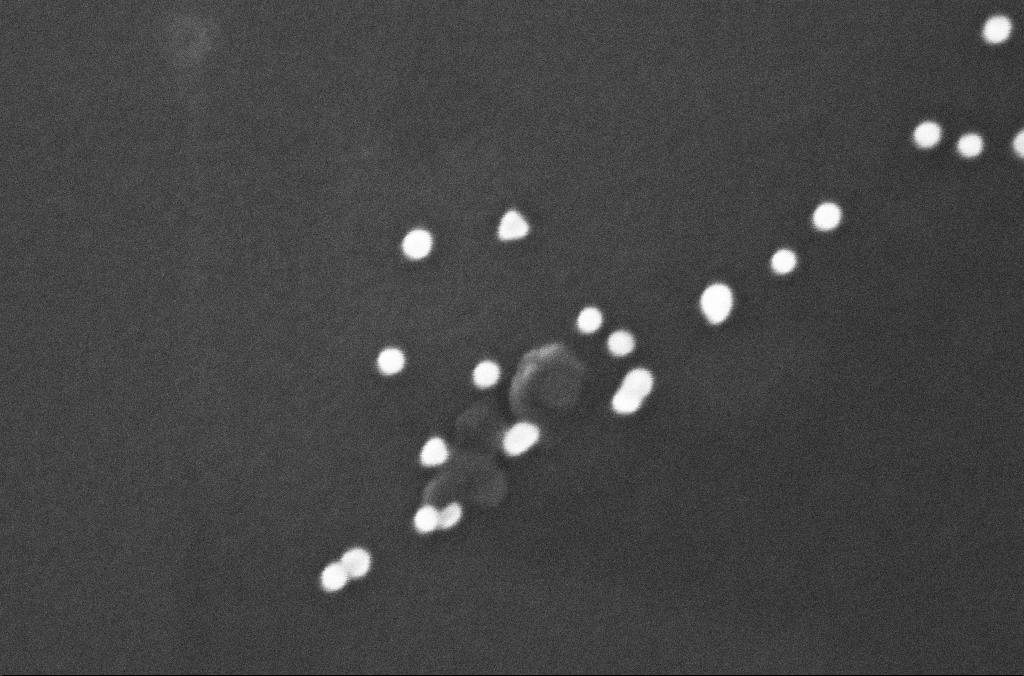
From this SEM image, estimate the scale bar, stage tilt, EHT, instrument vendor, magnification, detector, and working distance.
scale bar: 100 nm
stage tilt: -0°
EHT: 10 kV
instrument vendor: Zeiss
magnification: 435.02 K X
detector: InLens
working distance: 2.5 mm